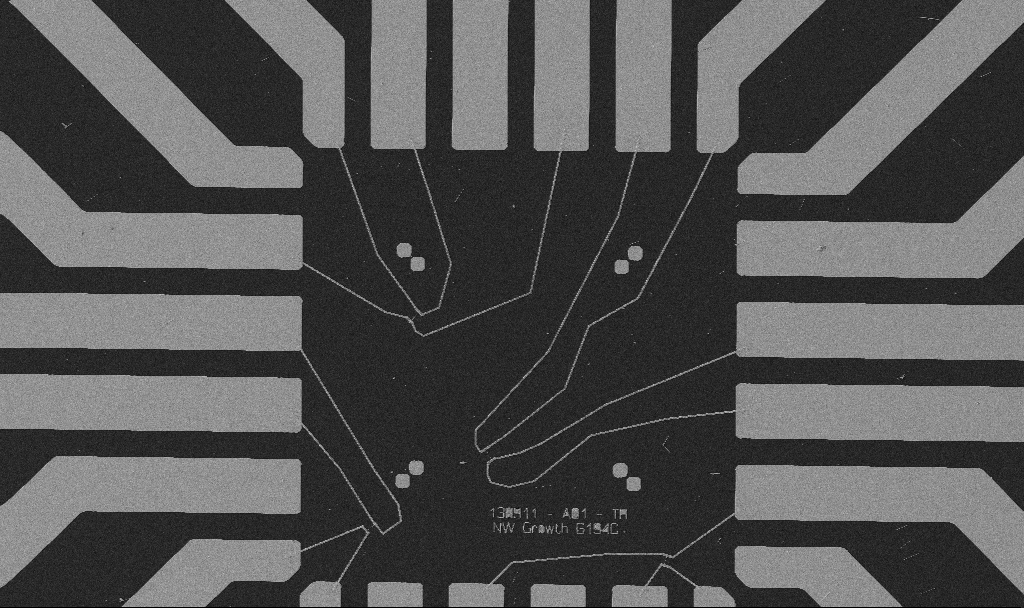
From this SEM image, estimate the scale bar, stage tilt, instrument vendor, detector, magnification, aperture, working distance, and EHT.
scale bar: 20000 nm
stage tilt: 0°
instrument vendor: Zeiss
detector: SE2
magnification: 1 K X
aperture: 30 µm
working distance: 10.7 mm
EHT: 5 kV